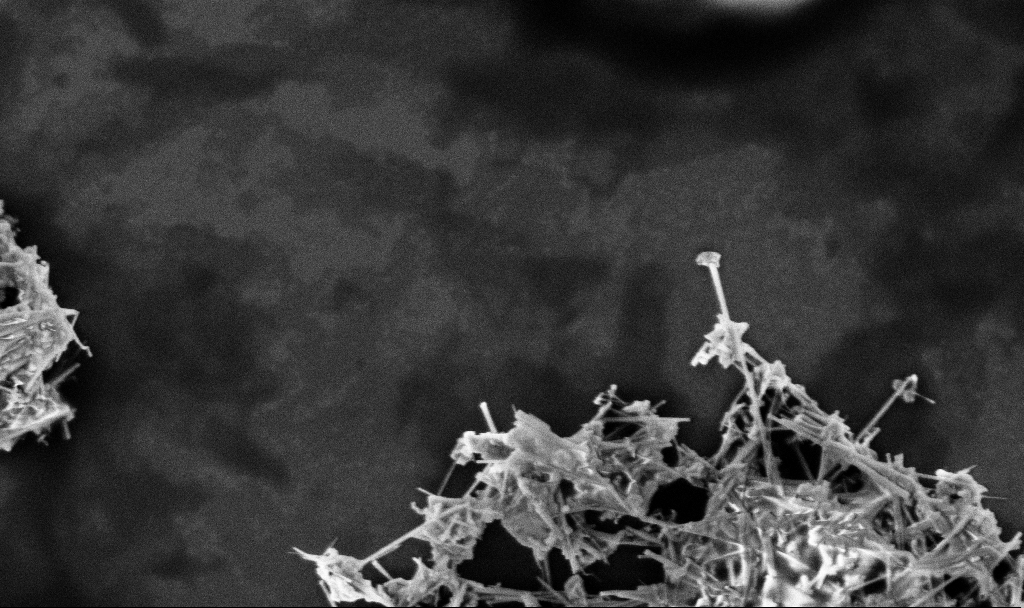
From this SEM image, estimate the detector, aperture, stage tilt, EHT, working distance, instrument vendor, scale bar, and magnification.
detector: InLens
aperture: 30 µm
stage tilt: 0°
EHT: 3 kV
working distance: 3.3 mm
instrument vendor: Zeiss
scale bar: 1000 nm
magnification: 37.2 K X